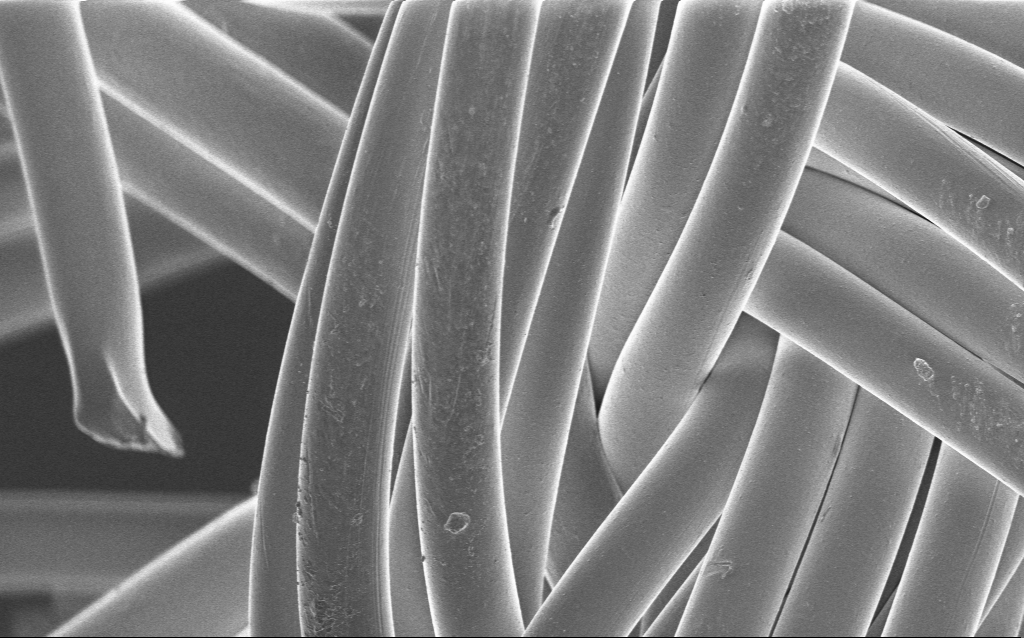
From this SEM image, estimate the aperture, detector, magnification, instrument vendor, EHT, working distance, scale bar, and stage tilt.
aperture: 30 µm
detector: InLens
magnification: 1.36 K X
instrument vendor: Zeiss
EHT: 1 kV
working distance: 4 mm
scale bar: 20000 nm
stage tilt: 0°